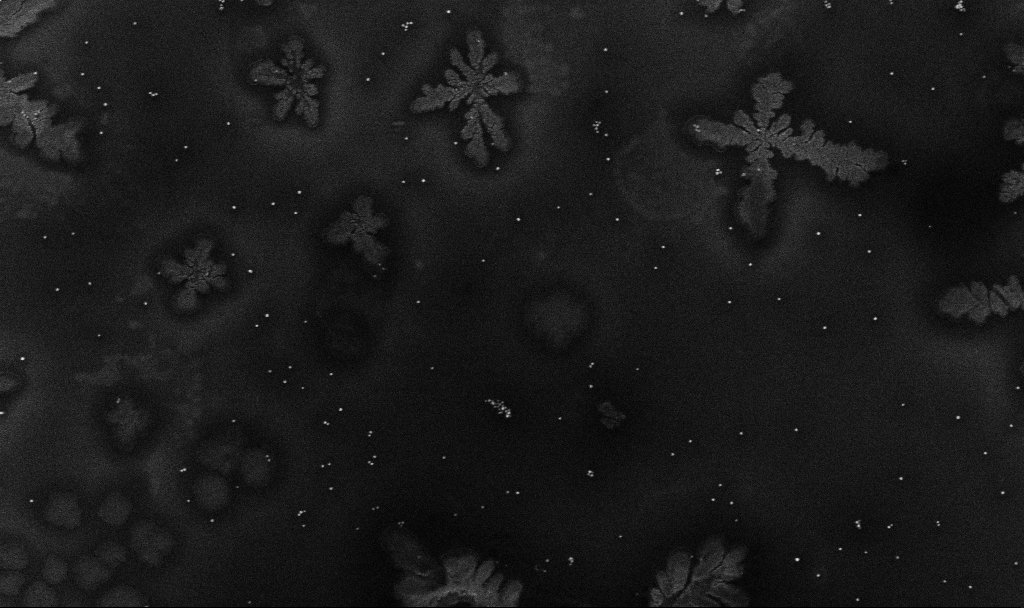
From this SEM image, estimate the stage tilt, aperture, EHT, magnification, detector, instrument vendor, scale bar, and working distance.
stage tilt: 0°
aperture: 30 µm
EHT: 10 kV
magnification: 50 K X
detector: InLens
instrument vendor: Zeiss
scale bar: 1000 nm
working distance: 3.3 mm